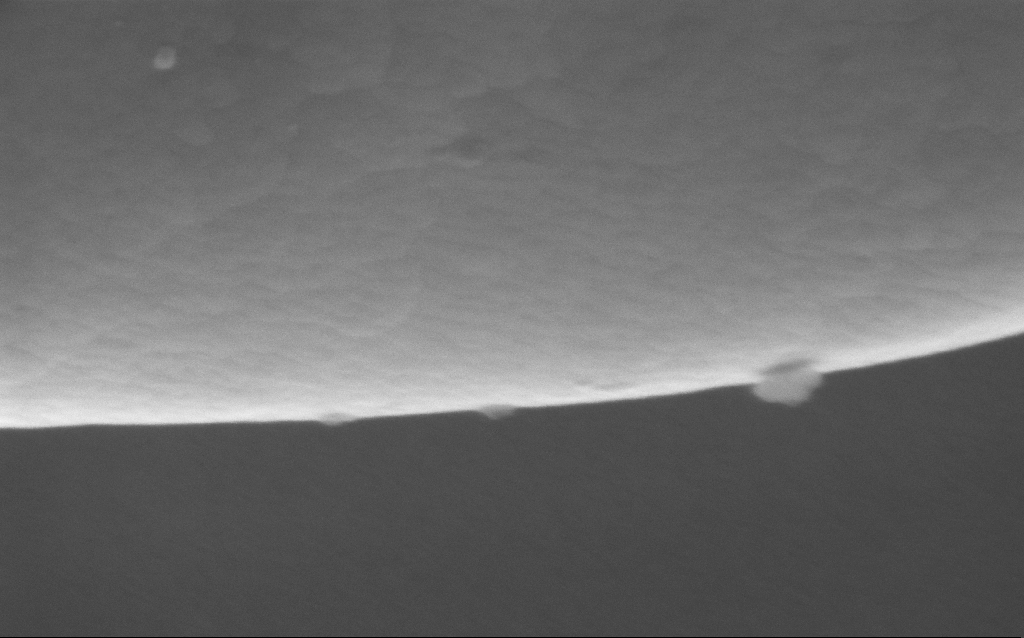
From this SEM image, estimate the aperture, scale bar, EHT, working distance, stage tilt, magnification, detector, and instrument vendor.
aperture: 30 µm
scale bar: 200 nm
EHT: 5 kV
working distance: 3 mm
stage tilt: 0°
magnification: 298.97 K X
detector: InLens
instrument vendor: Zeiss